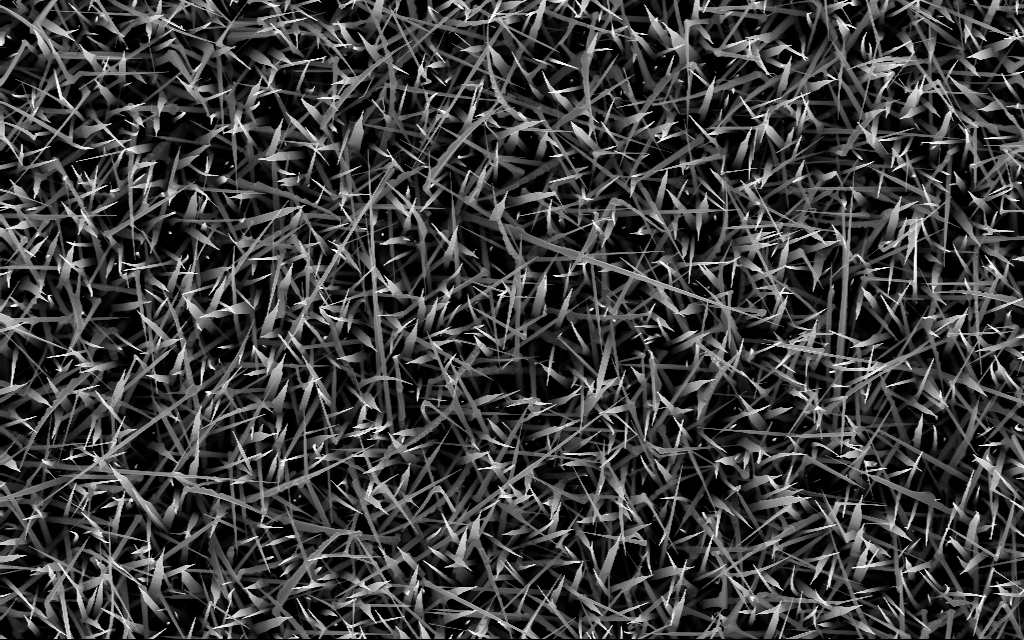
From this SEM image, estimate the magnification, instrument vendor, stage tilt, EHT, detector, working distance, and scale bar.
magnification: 10 K X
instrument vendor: Zeiss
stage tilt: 0°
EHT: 10 kV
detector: InLens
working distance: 7 mm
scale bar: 2000 nm